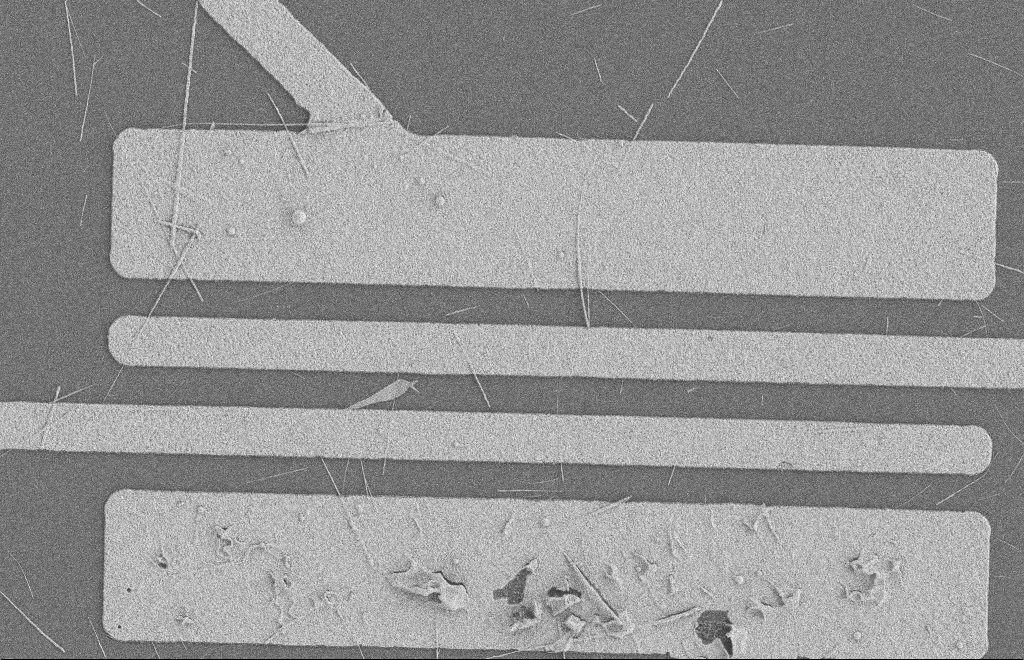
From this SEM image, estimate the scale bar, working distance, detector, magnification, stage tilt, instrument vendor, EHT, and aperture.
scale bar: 2000 nm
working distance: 8 mm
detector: SE2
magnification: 5.33 K X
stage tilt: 0°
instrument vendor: Zeiss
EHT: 2 kV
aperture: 20 µm